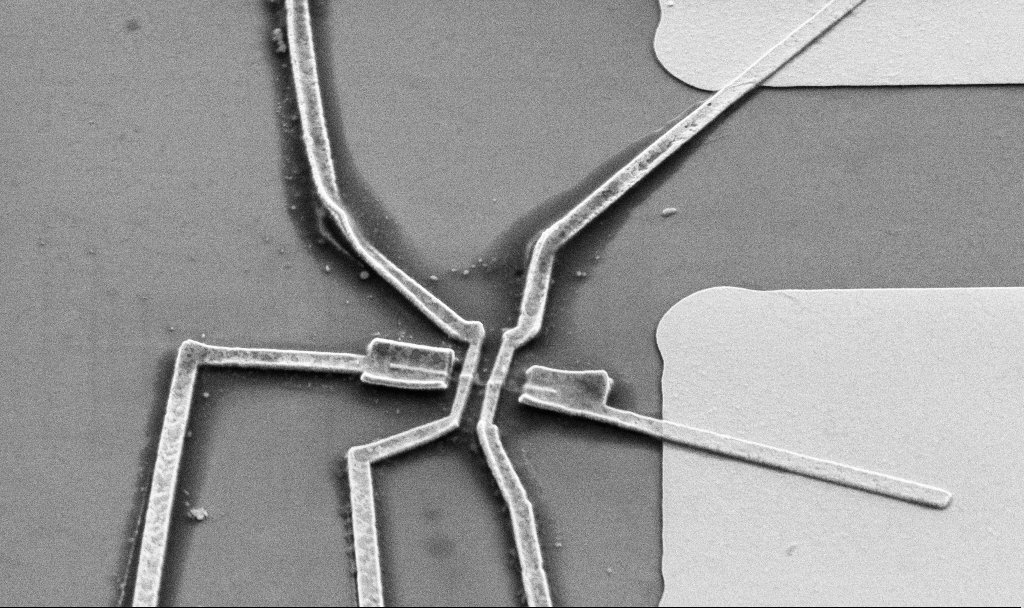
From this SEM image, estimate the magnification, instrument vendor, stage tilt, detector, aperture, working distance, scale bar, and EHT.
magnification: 10 K X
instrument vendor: Zeiss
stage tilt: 45°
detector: SE2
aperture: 30 µm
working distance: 12.4 mm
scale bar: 2000 nm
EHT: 5 kV